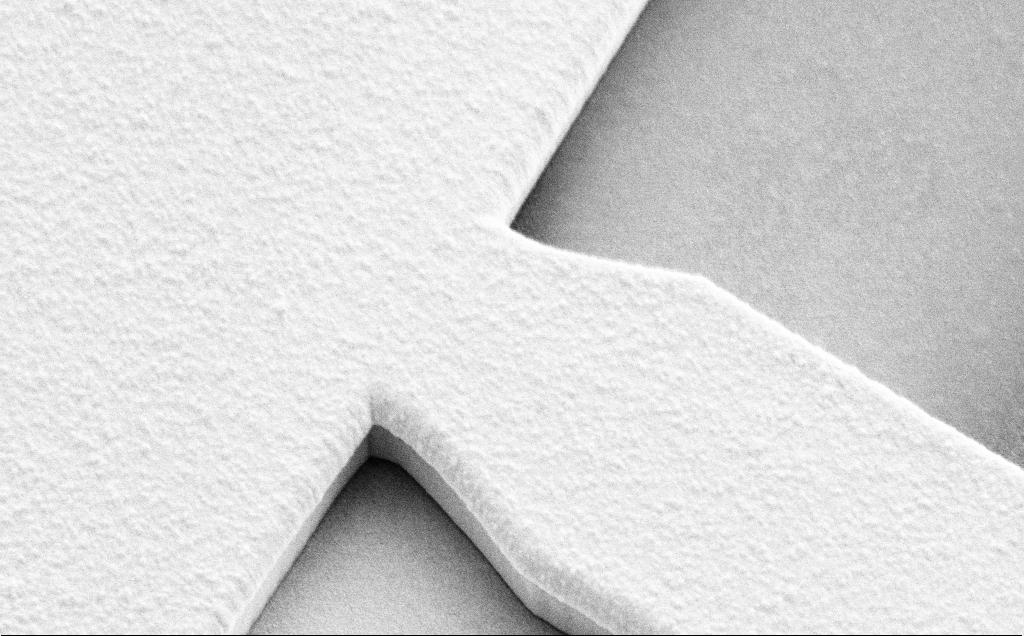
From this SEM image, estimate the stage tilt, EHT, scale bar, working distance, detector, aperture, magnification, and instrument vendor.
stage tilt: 45°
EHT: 10 kV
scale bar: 2000 nm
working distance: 12 mm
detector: SE2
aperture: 30 µm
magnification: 11.07 K X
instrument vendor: Zeiss